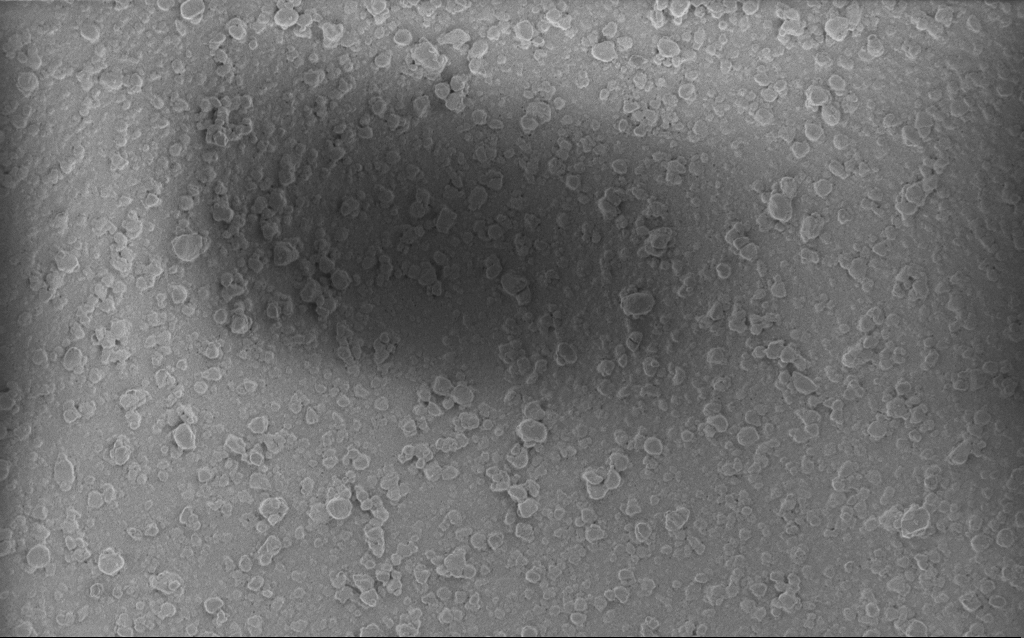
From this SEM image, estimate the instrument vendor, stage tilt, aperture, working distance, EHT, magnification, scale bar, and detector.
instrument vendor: Zeiss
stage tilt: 0°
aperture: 20 µm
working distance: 2.6 mm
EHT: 3 kV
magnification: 0.792 K X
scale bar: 20000 nm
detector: InLens